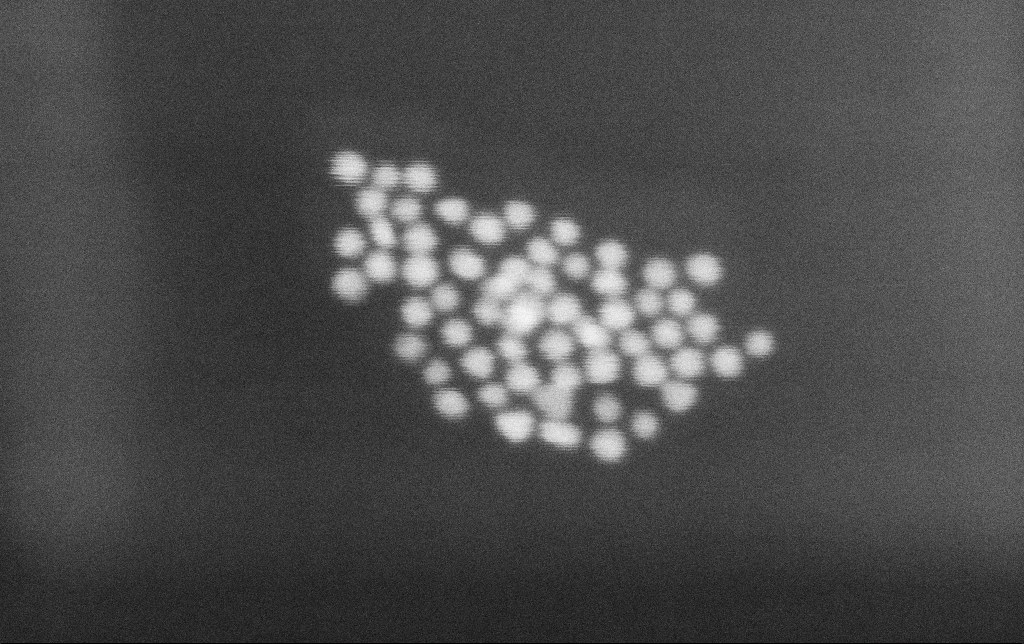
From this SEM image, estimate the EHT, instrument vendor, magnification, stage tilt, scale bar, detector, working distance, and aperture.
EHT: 30 kV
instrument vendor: Zeiss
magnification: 1000 K X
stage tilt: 0°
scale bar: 20 nm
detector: SE2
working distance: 10.8 mm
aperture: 30 µm